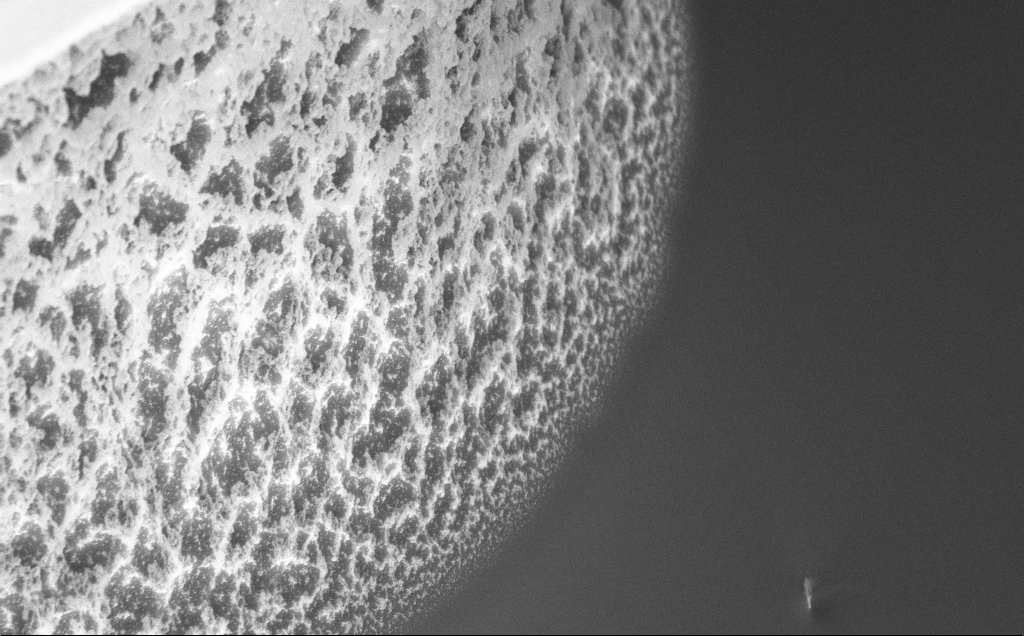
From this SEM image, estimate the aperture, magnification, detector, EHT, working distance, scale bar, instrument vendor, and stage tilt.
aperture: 30 µm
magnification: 24.74 K X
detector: InLens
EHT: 5 kV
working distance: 8 mm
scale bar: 2000 nm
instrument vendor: Zeiss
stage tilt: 45°